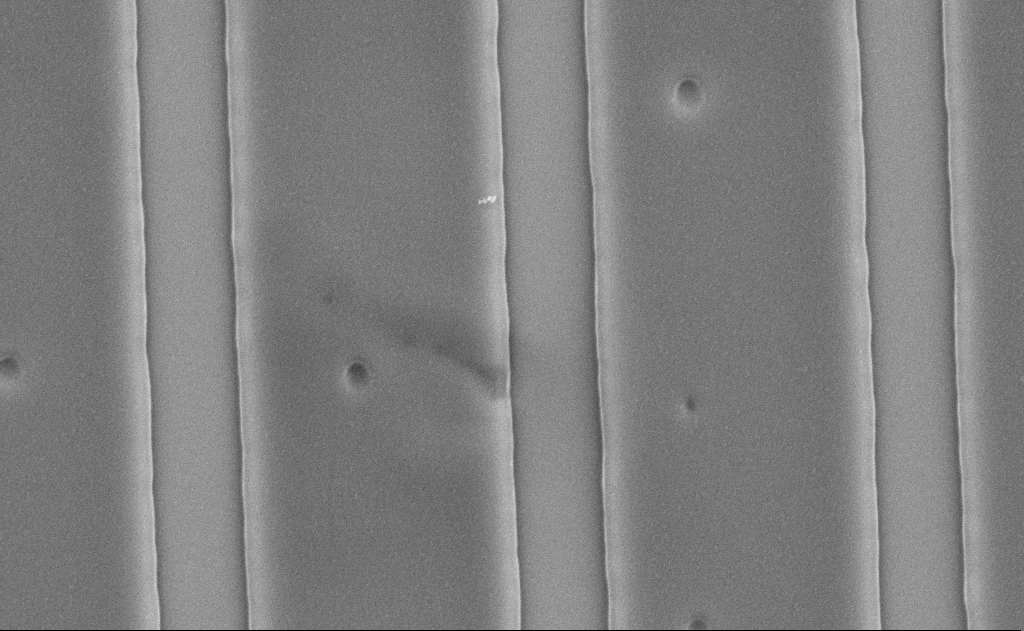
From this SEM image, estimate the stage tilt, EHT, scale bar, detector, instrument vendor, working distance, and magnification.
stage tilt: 0°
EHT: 5 kV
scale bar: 1000 nm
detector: SE2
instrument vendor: Zeiss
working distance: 12 mm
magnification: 32.84 K X